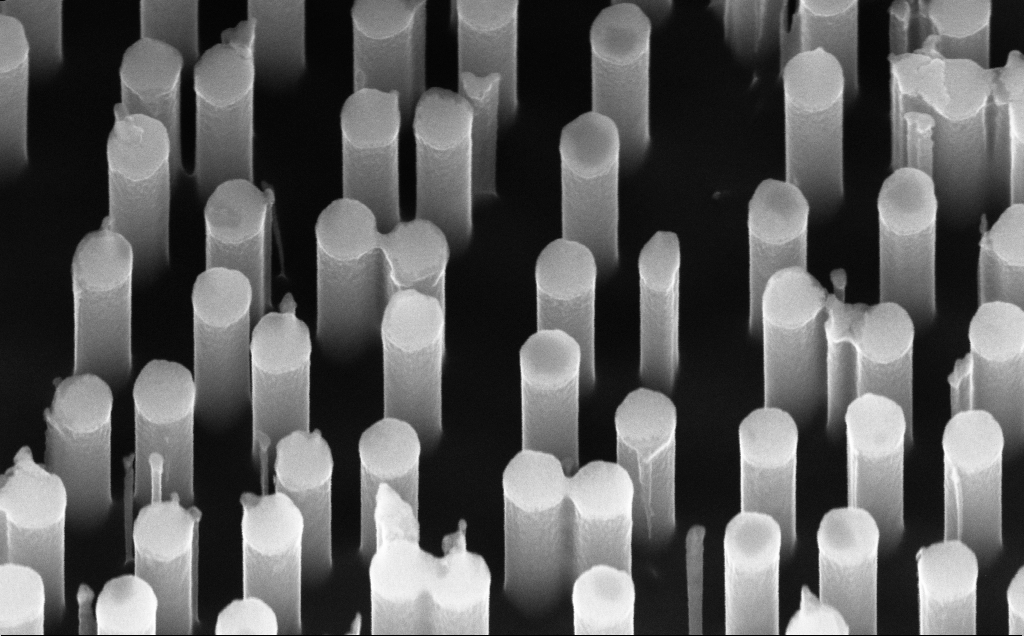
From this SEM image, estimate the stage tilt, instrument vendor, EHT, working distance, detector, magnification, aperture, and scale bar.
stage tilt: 45°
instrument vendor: Zeiss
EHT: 10 kV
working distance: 6 mm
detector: InLens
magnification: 104.61 K X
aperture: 30 µm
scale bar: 200 nm